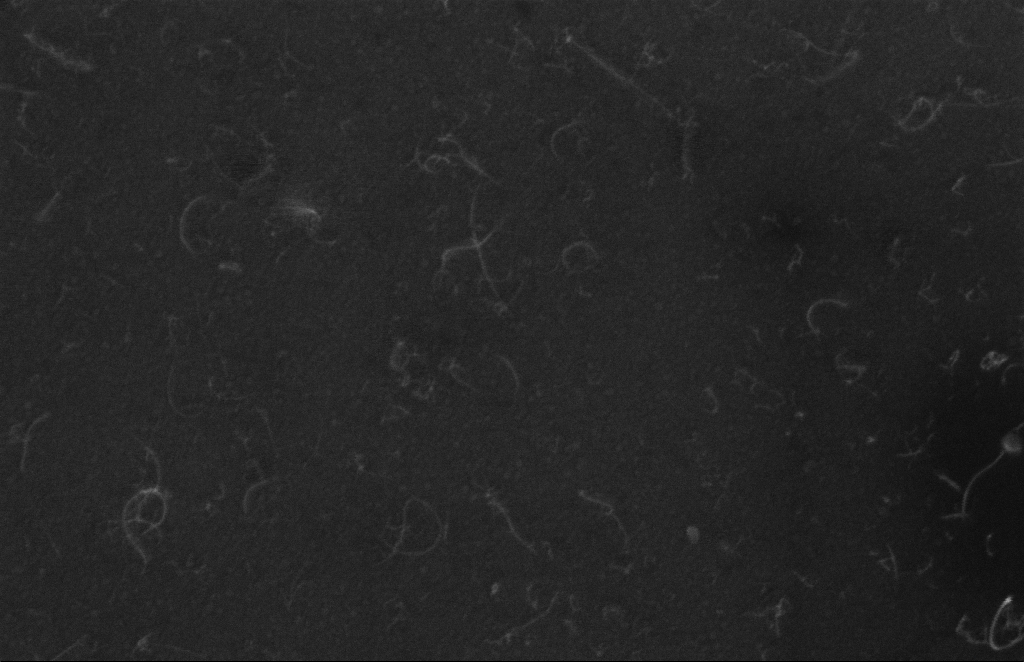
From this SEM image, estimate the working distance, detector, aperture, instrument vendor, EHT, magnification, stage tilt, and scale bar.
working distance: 12 mm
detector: InLens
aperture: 20 µm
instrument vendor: Zeiss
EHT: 5 kV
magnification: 127.02 K X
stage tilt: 0°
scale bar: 200 nm